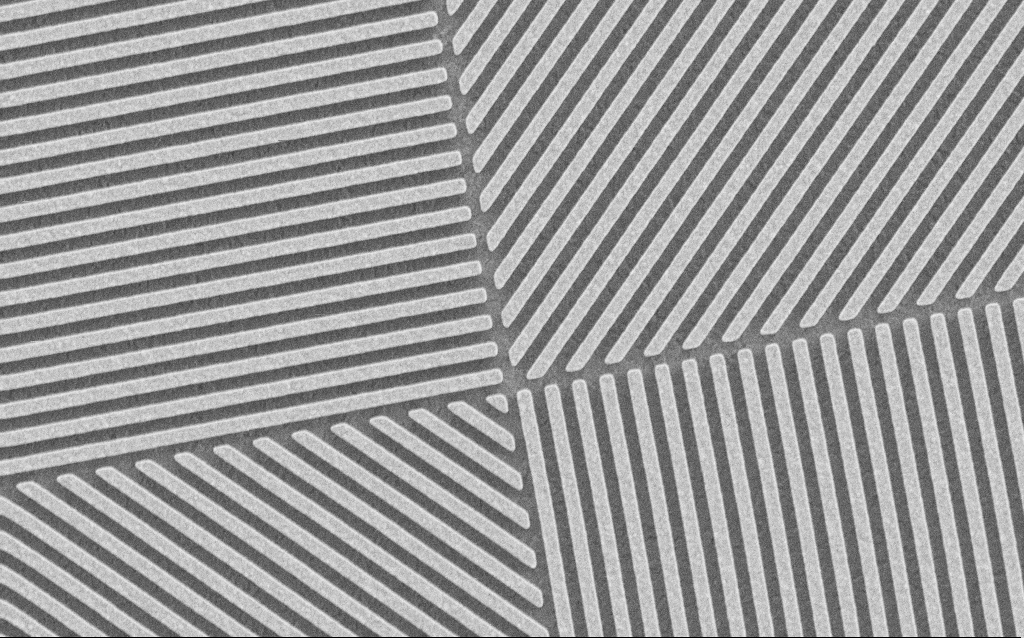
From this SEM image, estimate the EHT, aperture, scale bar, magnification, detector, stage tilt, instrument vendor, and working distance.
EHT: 3 kV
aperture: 30 µm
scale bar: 2000 nm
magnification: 25.92 K X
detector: SE2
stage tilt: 0°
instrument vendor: Zeiss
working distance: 4.5 mm